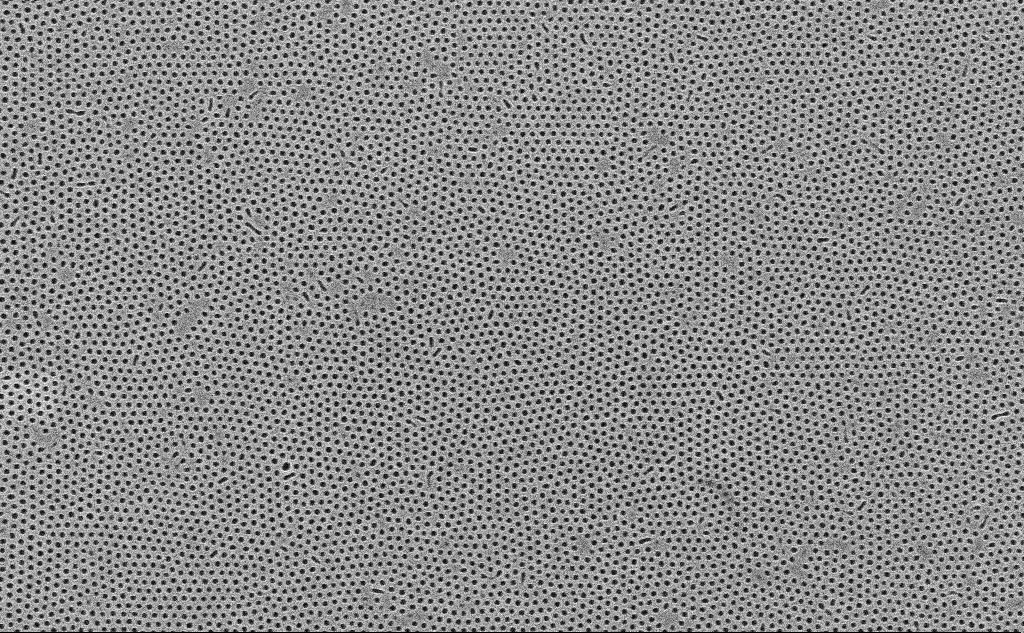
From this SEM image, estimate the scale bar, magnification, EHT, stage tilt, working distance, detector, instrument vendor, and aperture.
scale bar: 200 nm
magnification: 81.42 K X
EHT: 10 kV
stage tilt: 0°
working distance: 4.2 mm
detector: InLens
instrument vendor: Zeiss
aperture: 30 µm